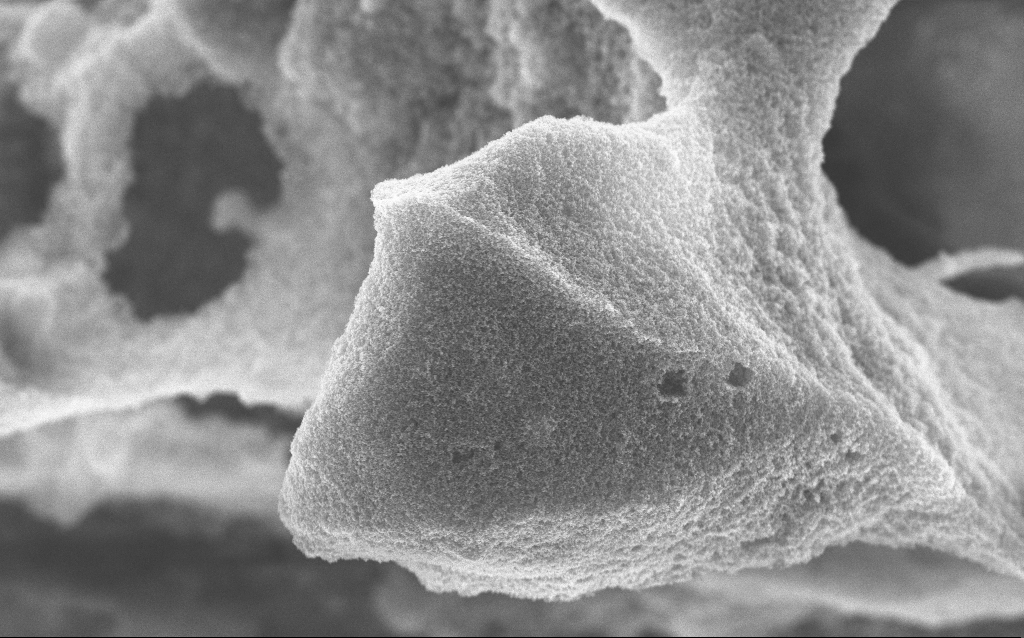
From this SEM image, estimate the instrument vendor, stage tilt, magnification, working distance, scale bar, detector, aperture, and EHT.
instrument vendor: Zeiss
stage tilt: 0°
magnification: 15.33 K X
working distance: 2.7 mm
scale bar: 1000 nm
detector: InLens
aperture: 30 µm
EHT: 10 kV